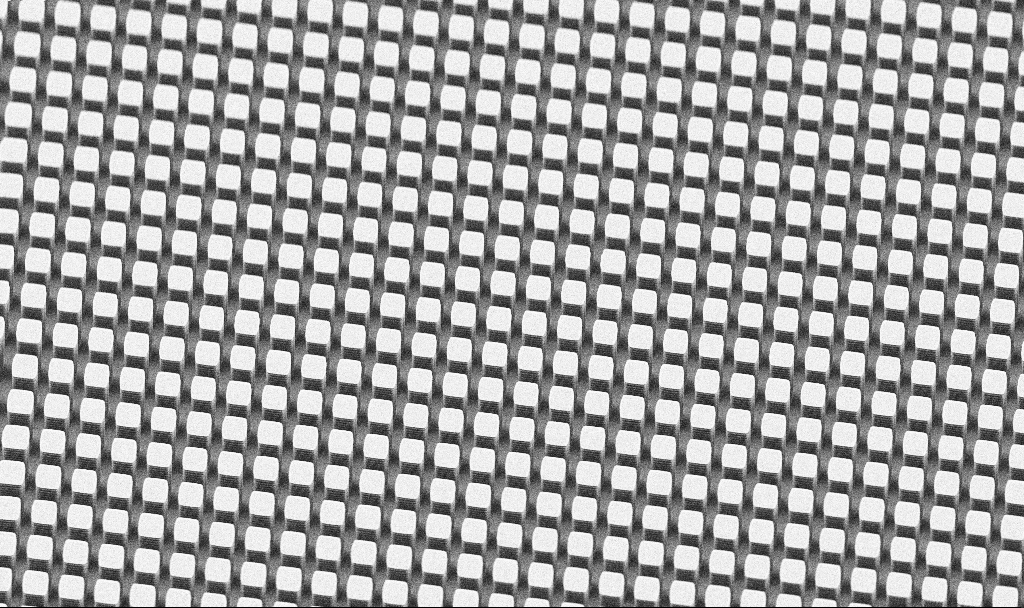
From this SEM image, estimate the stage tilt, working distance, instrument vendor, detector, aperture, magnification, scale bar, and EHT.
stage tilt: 45°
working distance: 7.5 mm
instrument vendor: Zeiss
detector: SE2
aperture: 30 µm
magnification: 2.19 K X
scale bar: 10000 nm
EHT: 5 kV